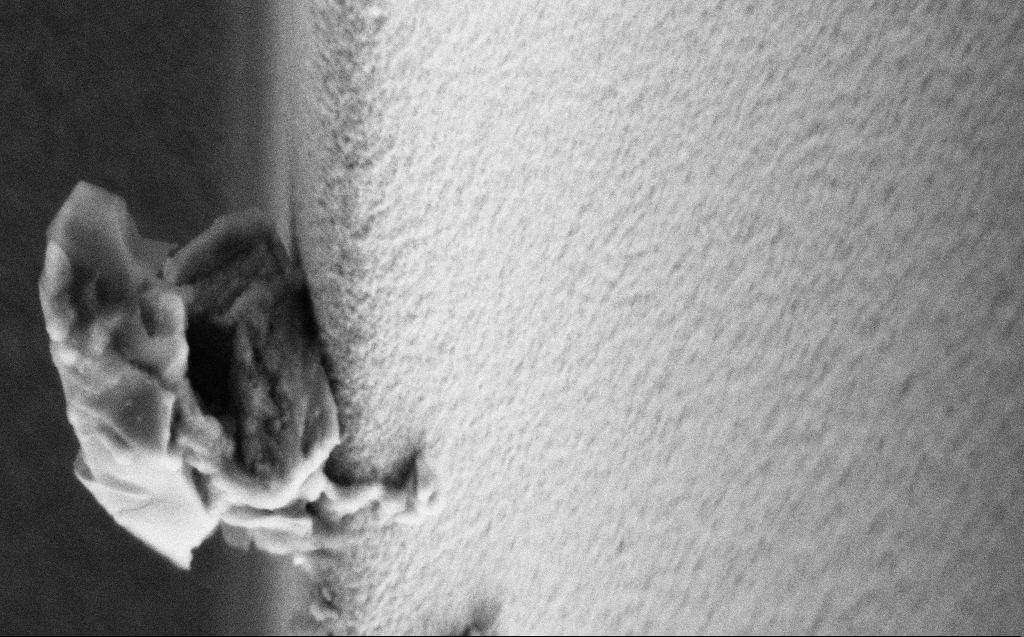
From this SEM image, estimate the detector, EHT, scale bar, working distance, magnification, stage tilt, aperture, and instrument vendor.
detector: SE2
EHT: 3 kV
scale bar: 1000 nm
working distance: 5 mm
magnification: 52.3 K X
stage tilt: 45°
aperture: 30 µm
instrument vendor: Zeiss